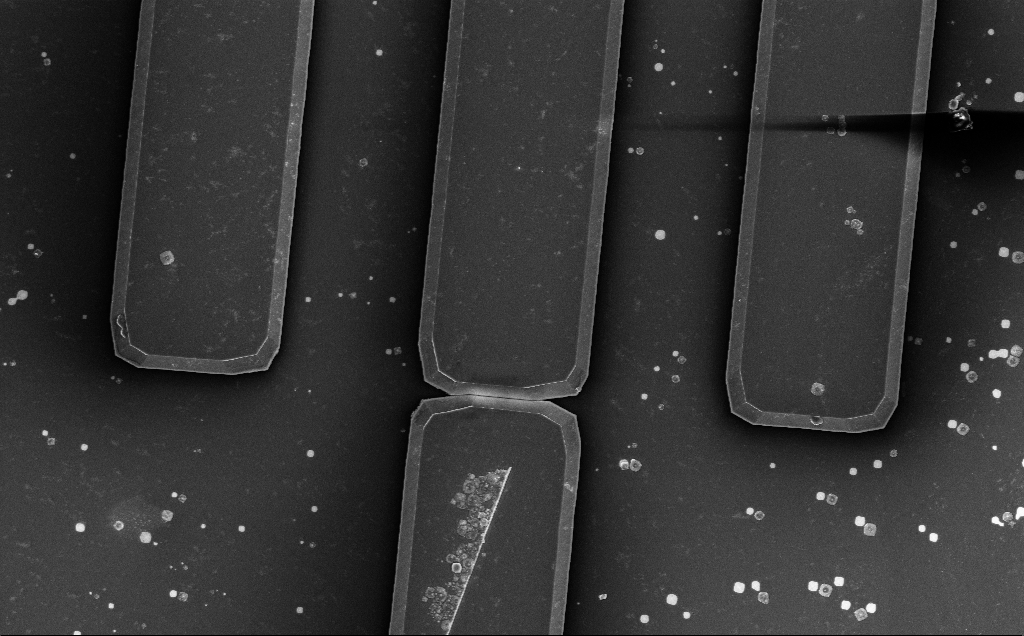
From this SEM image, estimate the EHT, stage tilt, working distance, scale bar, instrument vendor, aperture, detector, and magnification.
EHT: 5 kV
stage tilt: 0°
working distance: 14 mm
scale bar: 10000 nm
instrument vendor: Zeiss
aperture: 30 µm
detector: InLens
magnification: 2.84 K X